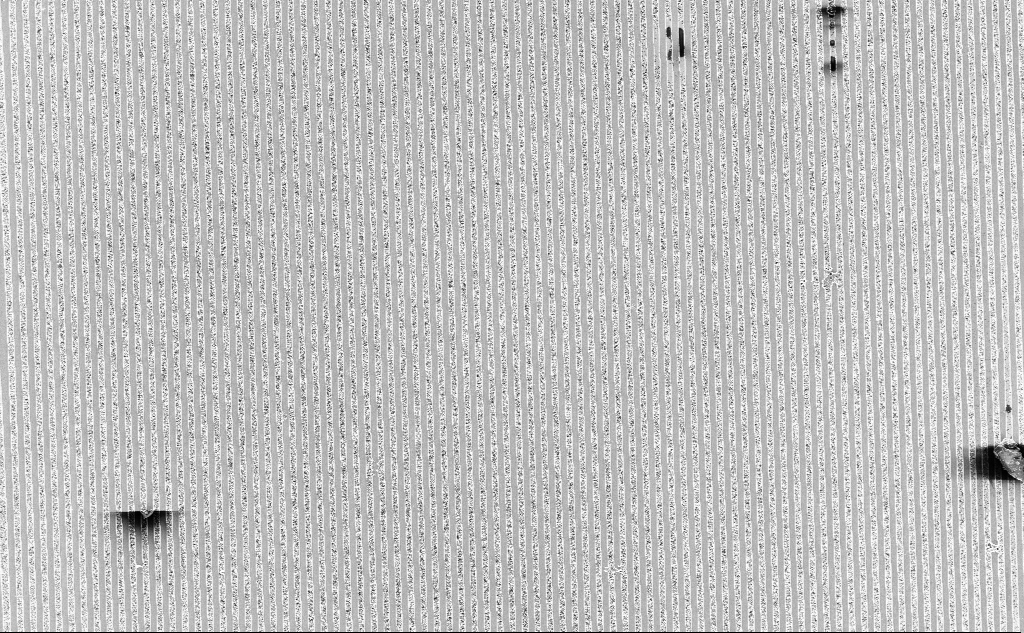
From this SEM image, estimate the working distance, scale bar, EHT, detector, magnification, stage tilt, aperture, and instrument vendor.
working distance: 7.9 mm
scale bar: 20000 nm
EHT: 3 kV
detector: InLens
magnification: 1.15 K X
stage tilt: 0°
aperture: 30 µm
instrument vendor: Zeiss